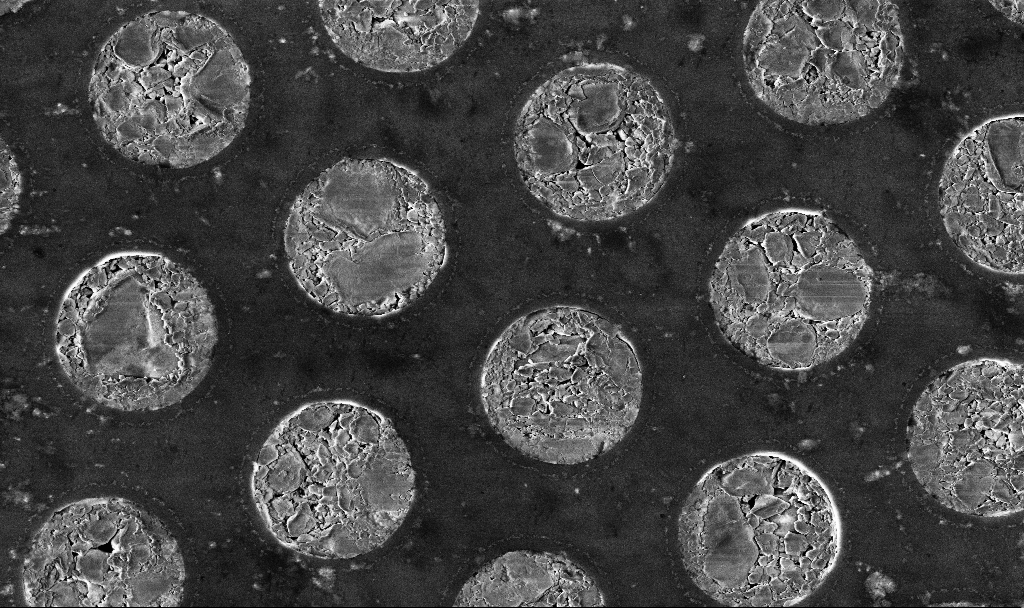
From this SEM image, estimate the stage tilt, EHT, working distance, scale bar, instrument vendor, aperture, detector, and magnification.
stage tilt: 0°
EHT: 3 kV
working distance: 4.8 mm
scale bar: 1000 nm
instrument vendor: Zeiss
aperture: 30 µm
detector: InLens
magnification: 15.1 K X